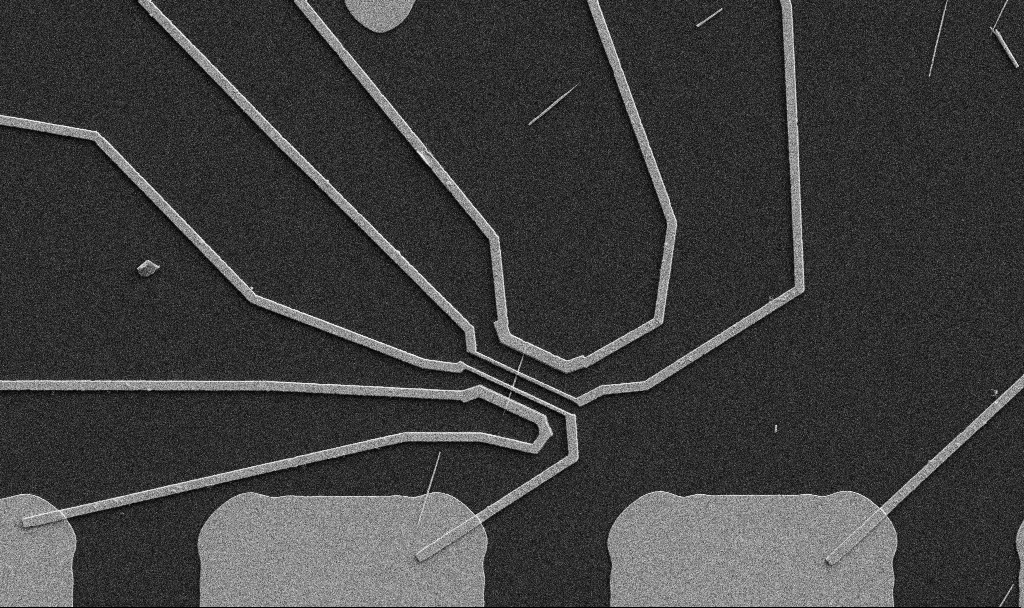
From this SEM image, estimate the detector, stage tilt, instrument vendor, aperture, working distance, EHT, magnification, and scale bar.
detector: SE2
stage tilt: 0°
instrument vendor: Zeiss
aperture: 30 µm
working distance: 10.7 mm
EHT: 5 kV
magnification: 5 K X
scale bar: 10000 nm